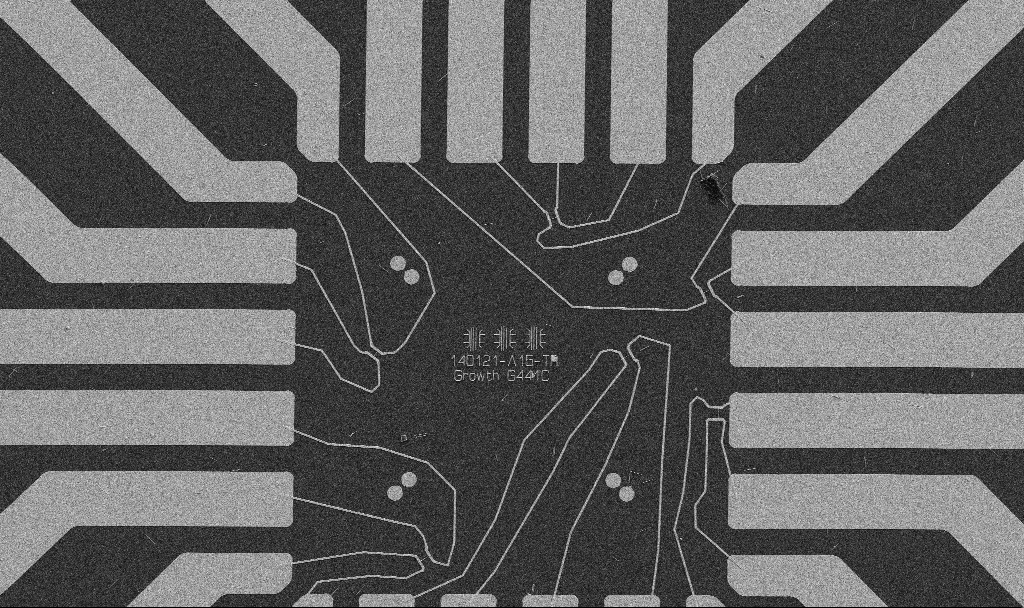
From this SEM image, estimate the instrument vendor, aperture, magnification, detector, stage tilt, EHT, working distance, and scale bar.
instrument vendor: Zeiss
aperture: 30 µm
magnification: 1 K X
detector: SE2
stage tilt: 0°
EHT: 5 kV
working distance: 10.7 mm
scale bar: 20000 nm